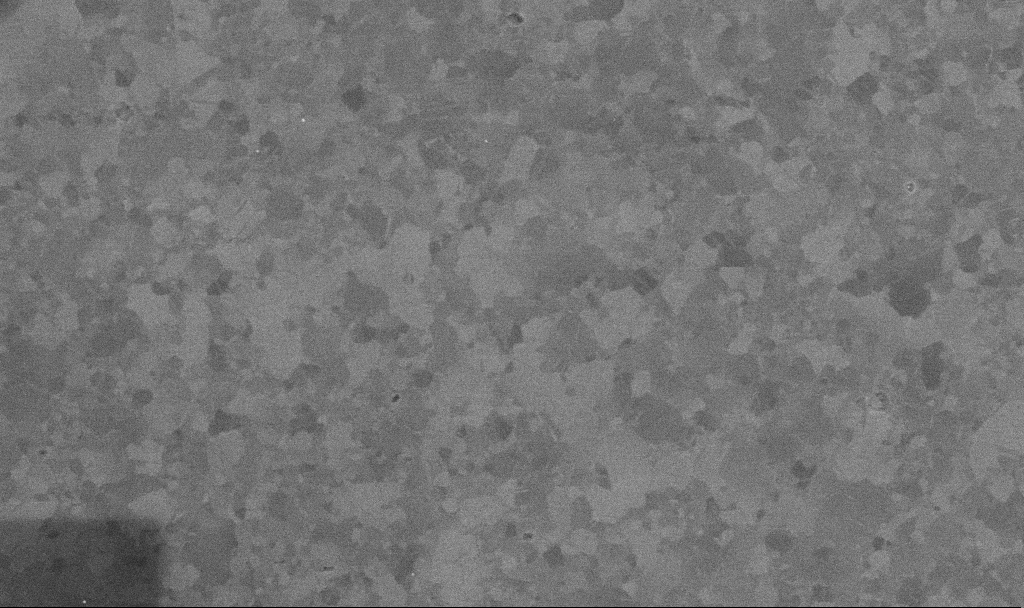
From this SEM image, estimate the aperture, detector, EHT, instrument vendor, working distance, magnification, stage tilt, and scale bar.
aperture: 30 µm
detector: InLens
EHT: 10 kV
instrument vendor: Zeiss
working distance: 3.3 mm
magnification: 50 K X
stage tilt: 0°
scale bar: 1000 nm